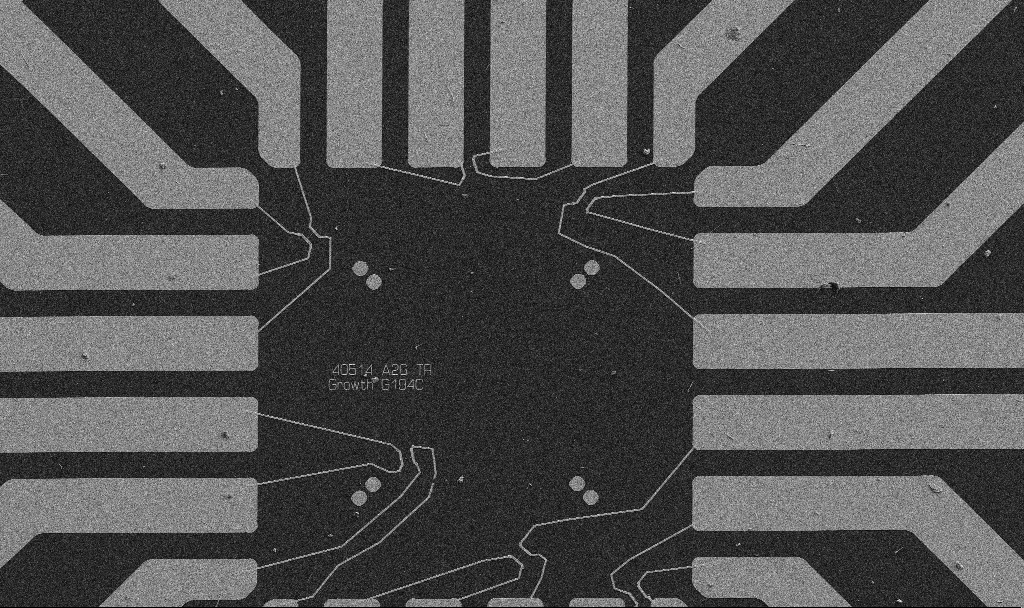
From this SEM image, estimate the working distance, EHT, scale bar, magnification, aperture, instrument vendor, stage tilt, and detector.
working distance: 10.7 mm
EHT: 5 kV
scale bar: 20000 nm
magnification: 1 K X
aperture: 30 µm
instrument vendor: Zeiss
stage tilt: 0°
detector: SE2